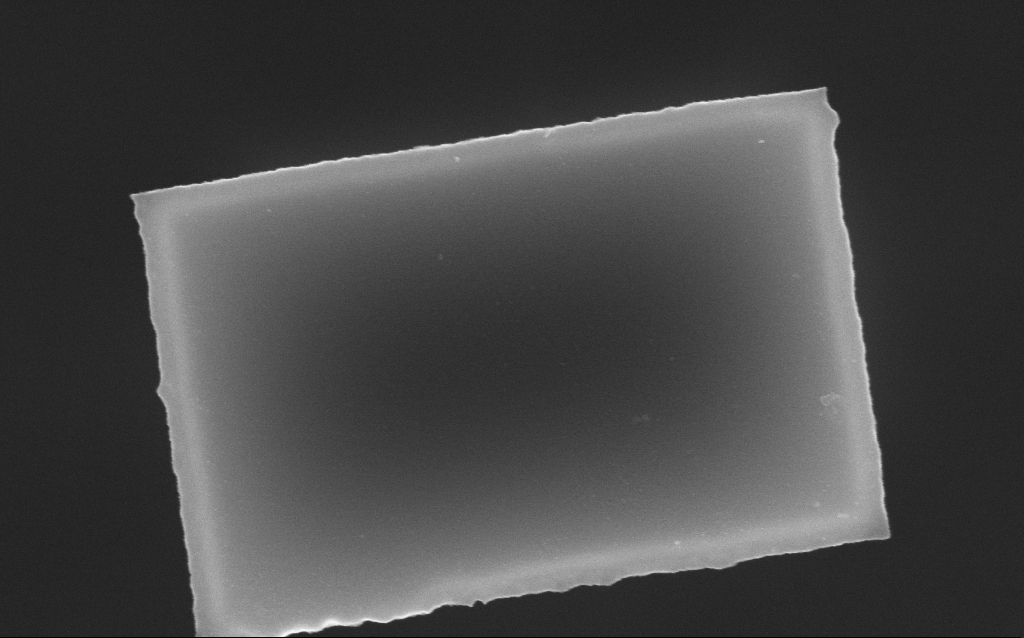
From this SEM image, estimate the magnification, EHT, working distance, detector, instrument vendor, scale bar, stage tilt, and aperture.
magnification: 87.01 K X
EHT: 10 kV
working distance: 8.4 mm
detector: InLens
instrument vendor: Zeiss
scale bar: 200 nm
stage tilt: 0°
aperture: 30 µm